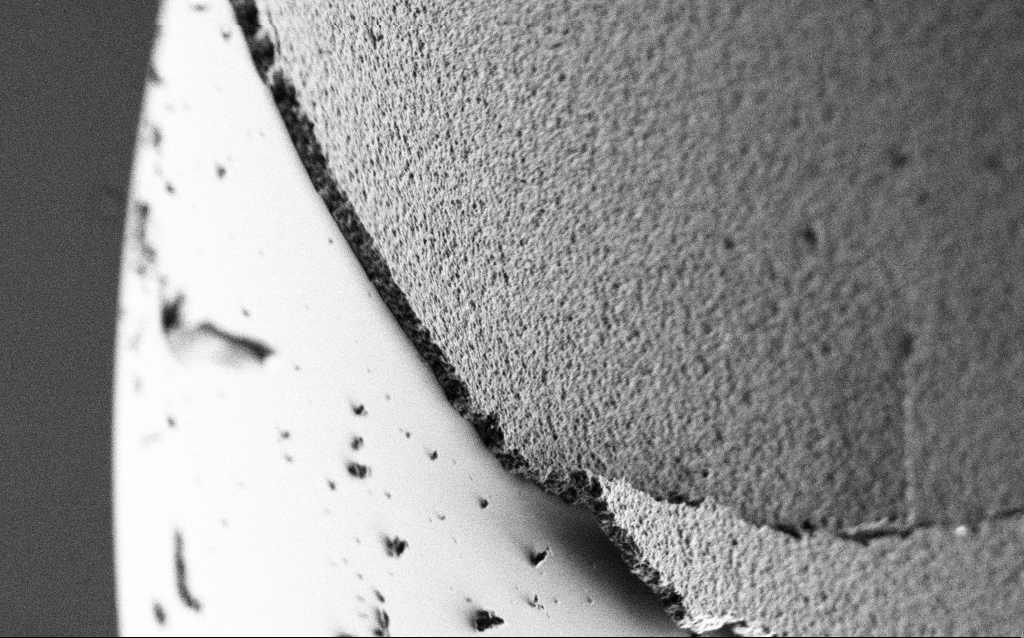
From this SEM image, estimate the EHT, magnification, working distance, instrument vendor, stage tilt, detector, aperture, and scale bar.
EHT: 1 kV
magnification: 22.27 K X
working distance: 5 mm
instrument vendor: Zeiss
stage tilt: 45°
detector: SE2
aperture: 30 µm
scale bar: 2000 nm